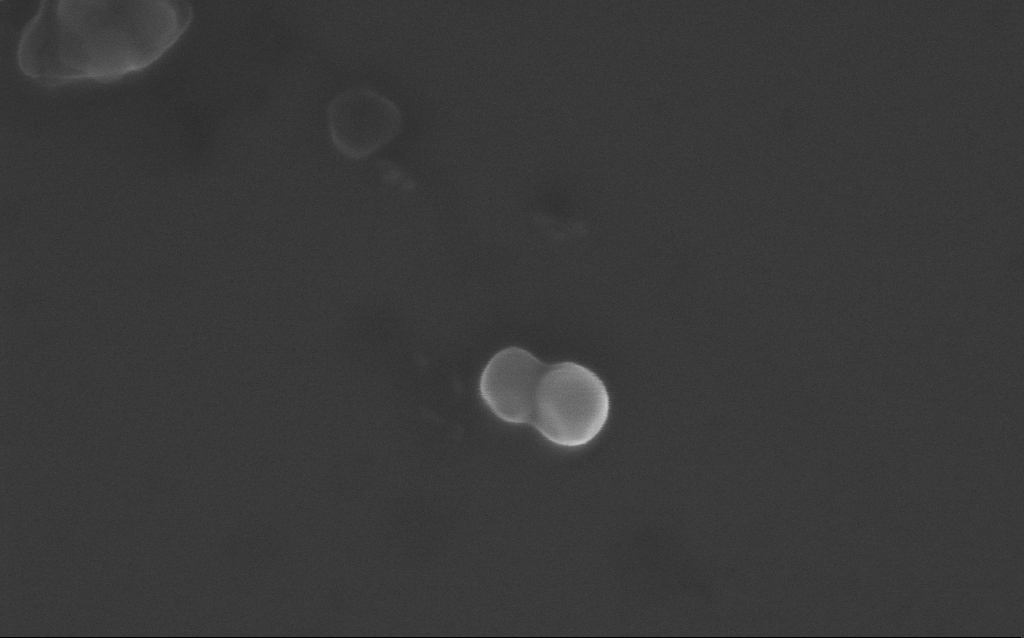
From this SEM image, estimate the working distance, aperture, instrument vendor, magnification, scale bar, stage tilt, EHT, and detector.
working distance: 3 mm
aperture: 30 µm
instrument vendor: Zeiss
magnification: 196.52 K X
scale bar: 200 nm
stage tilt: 0°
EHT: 10 kV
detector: InLens